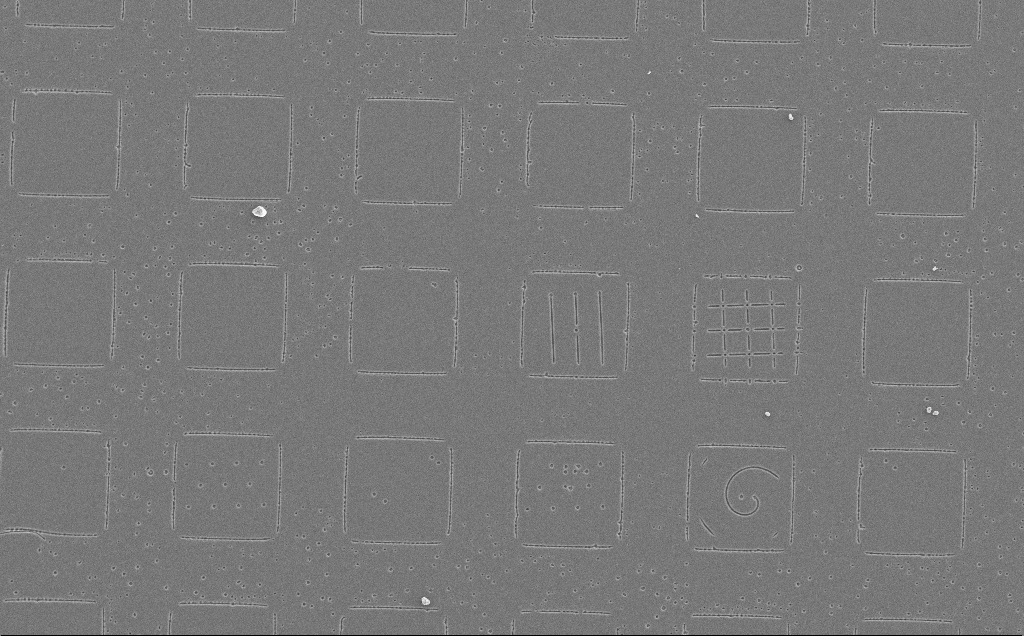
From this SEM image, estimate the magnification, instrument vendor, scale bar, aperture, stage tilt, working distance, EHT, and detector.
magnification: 0.448 K X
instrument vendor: Zeiss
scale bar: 100000 nm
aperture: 30 µm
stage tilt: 0°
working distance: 13 mm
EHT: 10 kV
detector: SE2